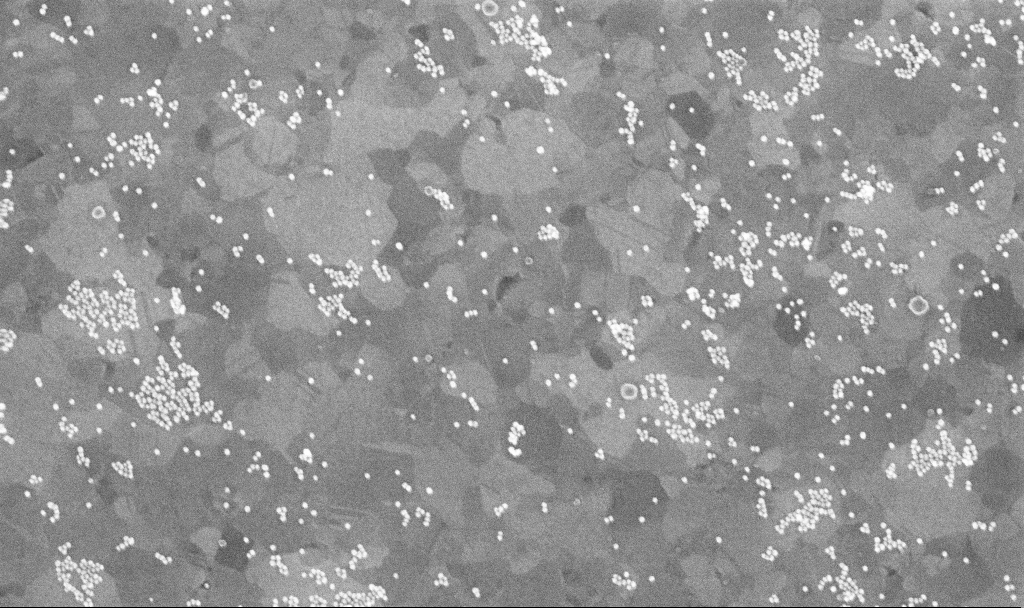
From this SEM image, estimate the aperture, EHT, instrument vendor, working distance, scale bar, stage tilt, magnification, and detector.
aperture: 30 µm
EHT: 10 kV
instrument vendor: Zeiss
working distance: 3.7 mm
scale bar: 200 nm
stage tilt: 0°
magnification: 100 K X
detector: InLens